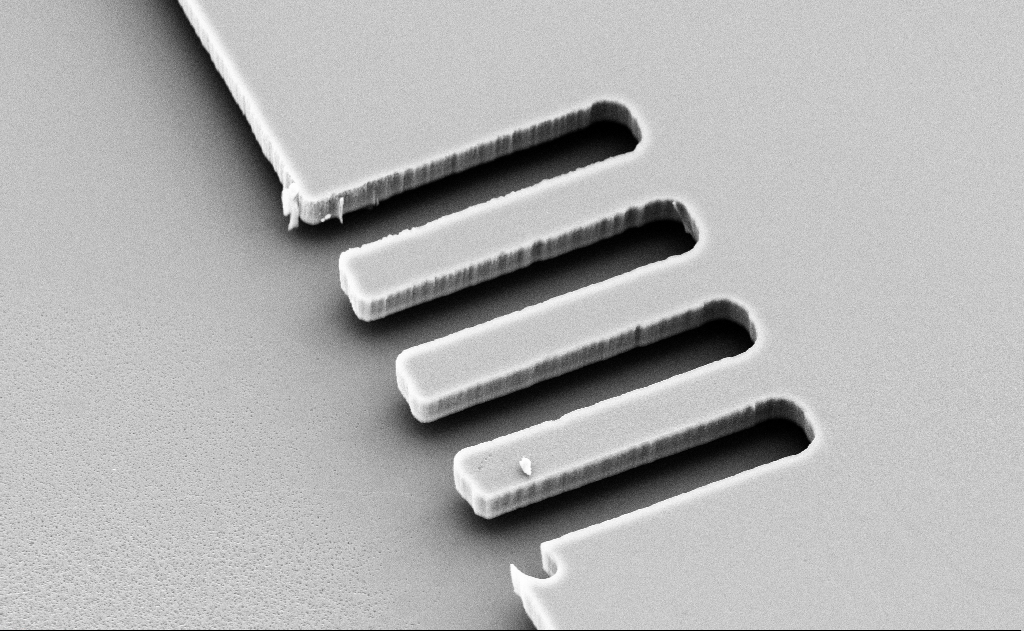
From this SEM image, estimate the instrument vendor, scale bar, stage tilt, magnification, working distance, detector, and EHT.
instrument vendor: Zeiss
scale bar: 10000 nm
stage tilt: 37.7°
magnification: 5.09 K X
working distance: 11 mm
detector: SE2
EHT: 10 kV